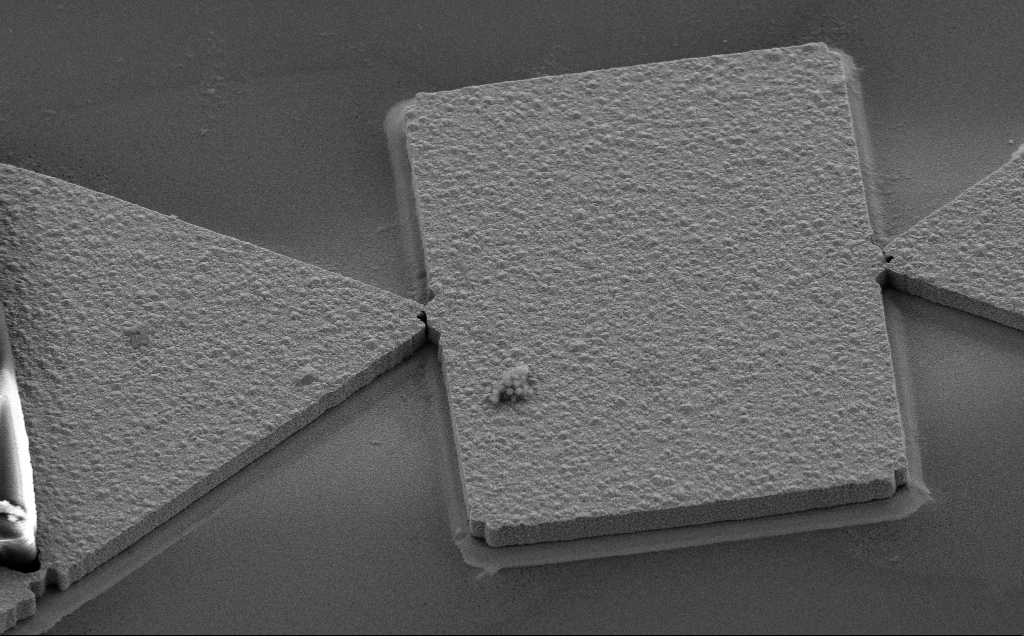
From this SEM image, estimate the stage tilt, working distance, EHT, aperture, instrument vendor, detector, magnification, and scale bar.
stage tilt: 35°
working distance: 8 mm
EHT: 10 kV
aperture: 30 µm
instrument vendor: Zeiss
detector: SE2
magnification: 3.27 K X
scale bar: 10000 nm